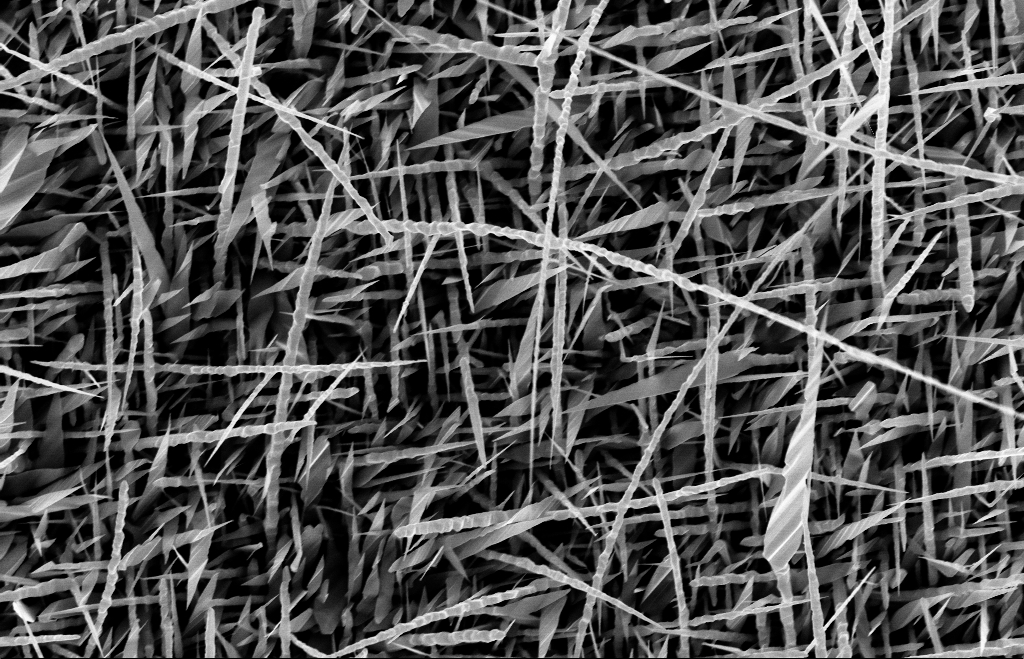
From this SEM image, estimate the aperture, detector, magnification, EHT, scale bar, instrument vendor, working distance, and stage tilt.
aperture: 30 µm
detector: InLens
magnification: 20 K X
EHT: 10 kV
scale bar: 1000 nm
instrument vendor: Zeiss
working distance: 8 mm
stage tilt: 0°